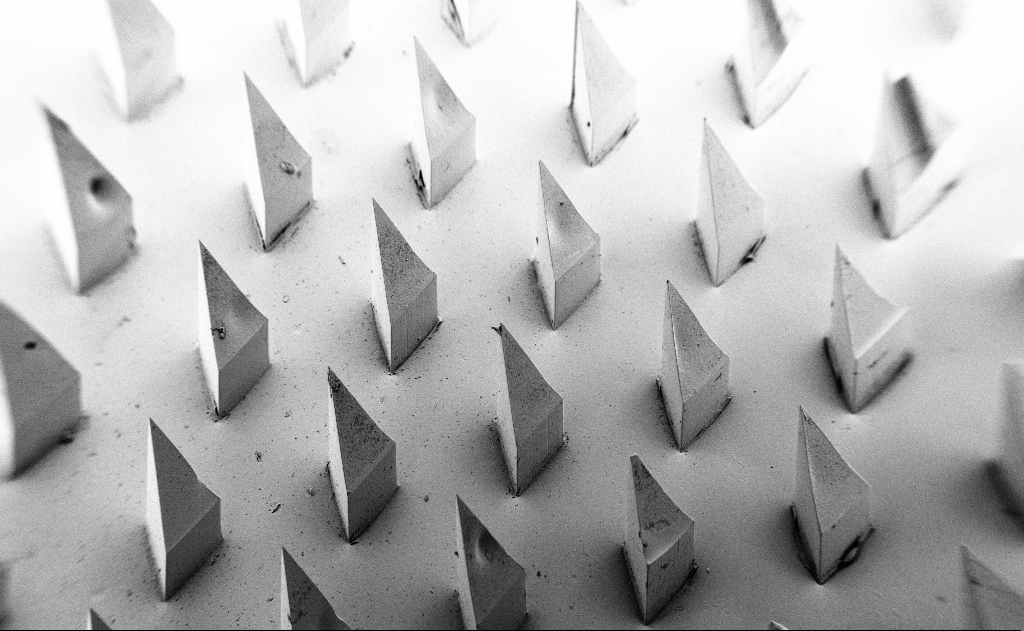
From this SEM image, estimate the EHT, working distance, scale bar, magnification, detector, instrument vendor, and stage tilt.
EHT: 5 kV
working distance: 9 mm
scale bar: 1e+06 nm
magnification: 0.067 K X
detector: SE2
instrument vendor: Zeiss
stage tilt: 45°